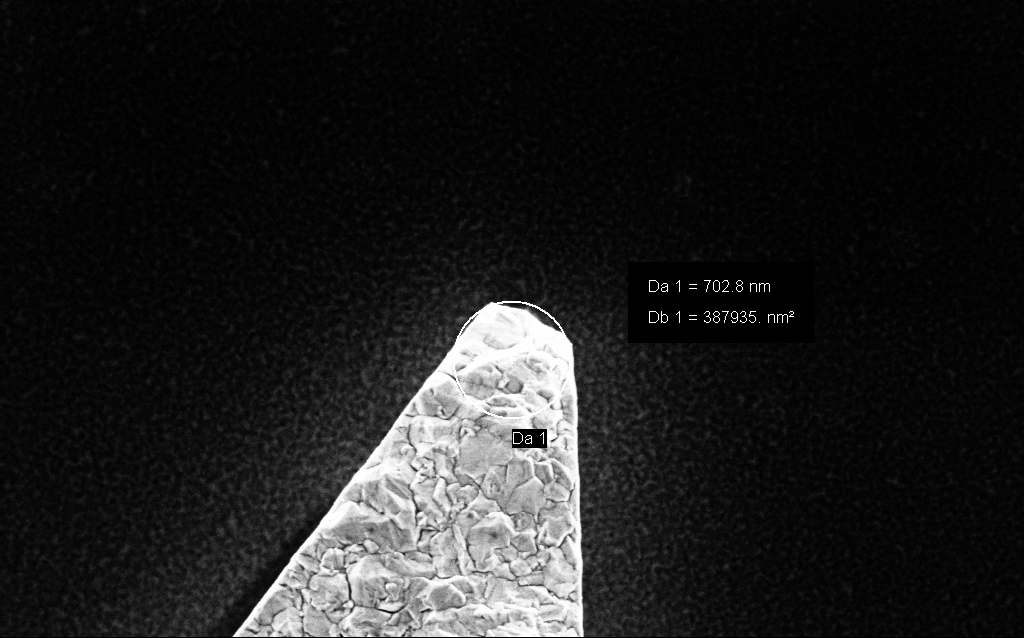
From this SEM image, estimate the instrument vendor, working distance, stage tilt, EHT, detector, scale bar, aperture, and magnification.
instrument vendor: Zeiss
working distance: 4.5 mm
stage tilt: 0°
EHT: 3 kV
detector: InLens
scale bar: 1000 nm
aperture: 30 µm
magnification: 61.65 K X